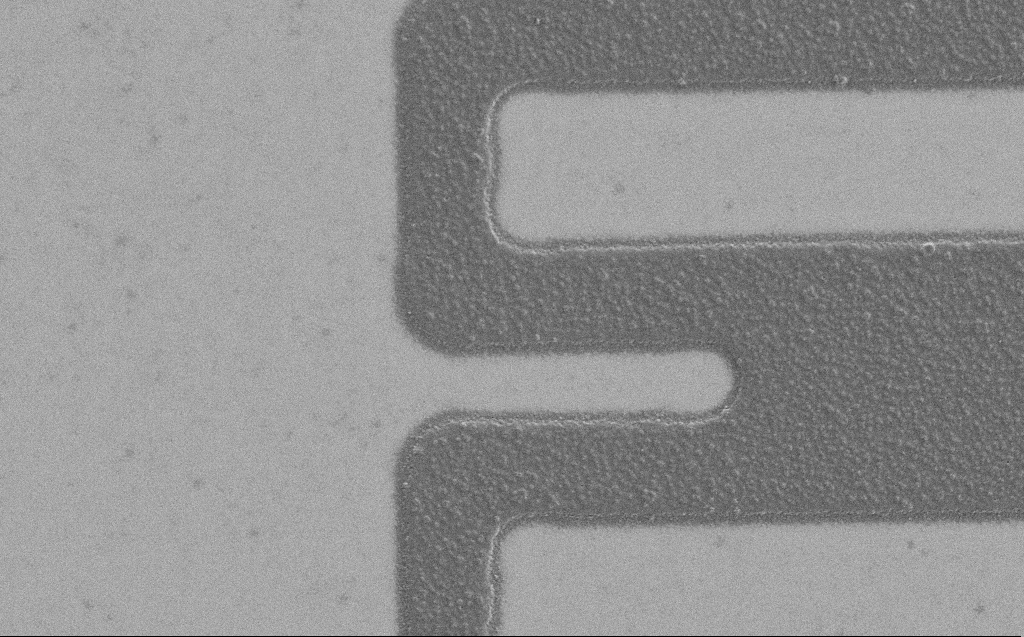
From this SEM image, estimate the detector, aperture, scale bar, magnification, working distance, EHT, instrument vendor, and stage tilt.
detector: SE2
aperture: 30 µm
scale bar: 10000 nm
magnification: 5.83 K X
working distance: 4 mm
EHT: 1.2 kV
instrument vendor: Zeiss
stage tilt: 0°